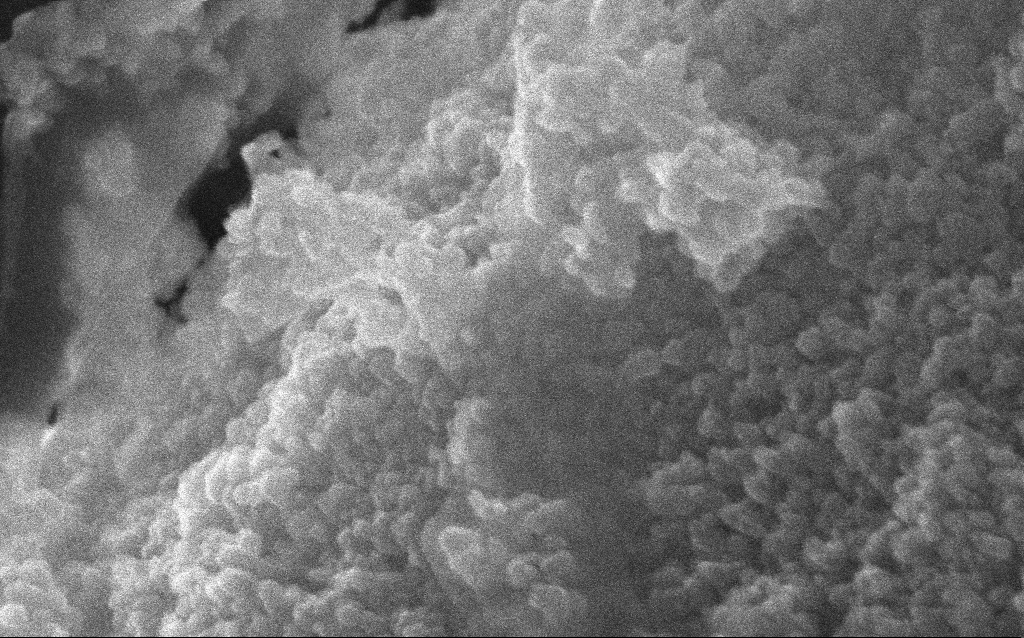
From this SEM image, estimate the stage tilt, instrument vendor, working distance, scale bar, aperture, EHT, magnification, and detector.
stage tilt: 0°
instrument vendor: Zeiss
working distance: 2.8 mm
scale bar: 100 nm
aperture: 30 µm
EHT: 10 kV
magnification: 348.1 K X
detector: InLens